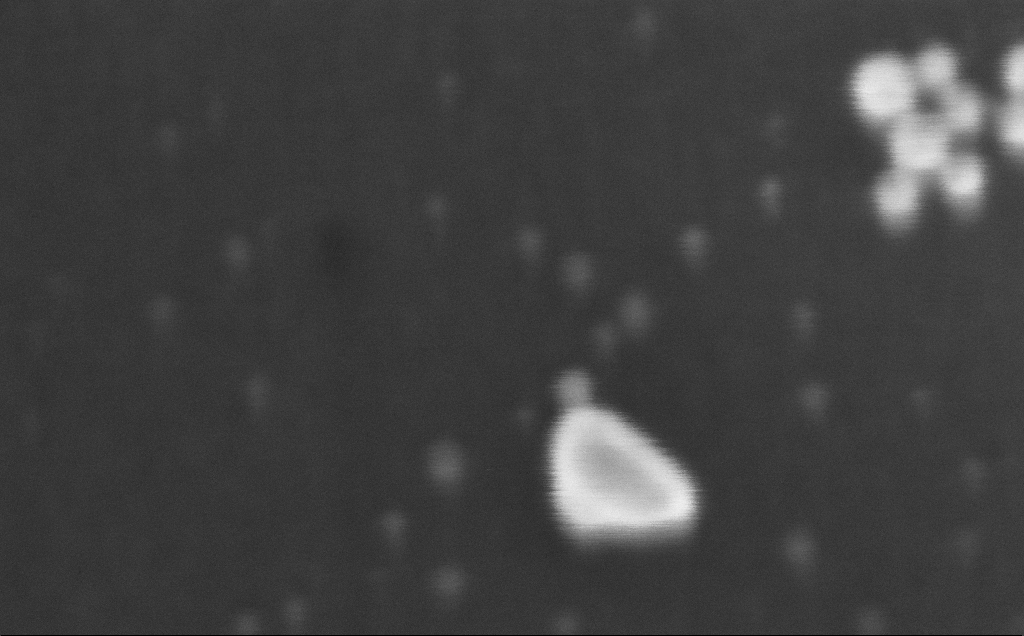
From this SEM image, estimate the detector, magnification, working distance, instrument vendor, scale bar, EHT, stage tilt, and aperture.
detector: InLens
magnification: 1717.56 K X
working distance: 3 mm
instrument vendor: Zeiss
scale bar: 20 nm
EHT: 20 kV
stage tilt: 0°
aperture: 30 µm